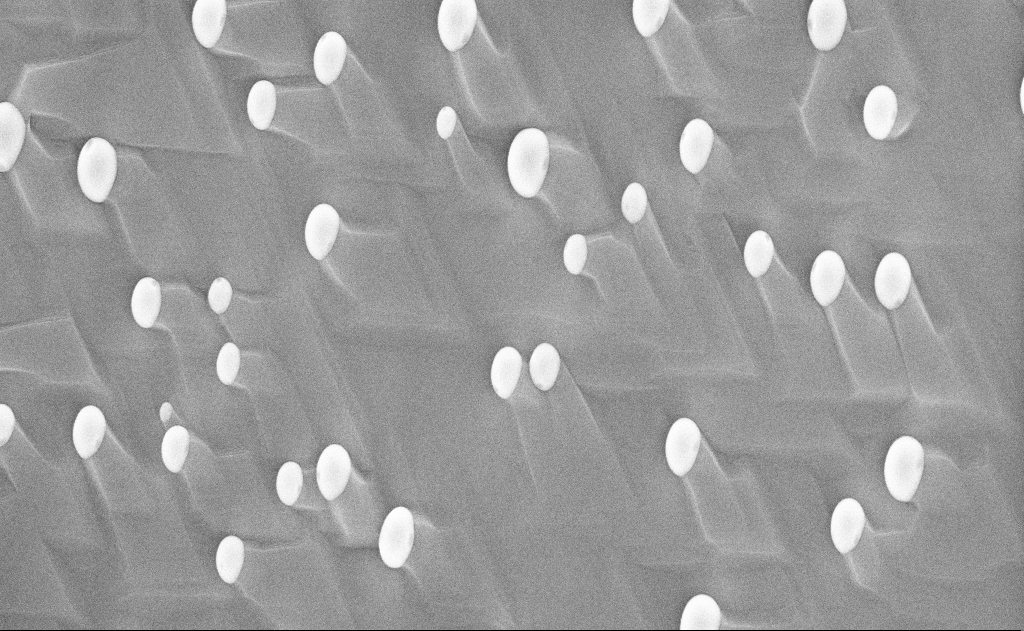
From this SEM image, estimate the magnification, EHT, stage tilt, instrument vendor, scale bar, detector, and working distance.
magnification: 20 K X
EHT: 10 kV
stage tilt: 0°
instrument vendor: Zeiss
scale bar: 2000 nm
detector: InLens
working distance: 12 mm